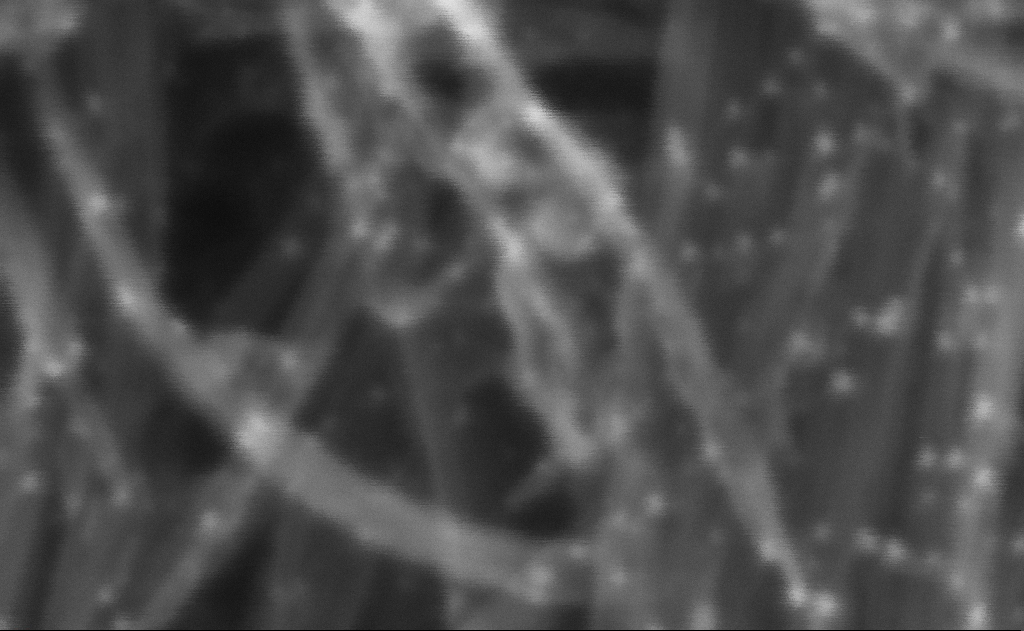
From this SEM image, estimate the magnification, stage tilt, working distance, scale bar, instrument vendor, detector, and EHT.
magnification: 2264.42 K X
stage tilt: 0°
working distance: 3 mm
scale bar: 20 nm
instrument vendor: Zeiss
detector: InLens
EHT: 10 kV